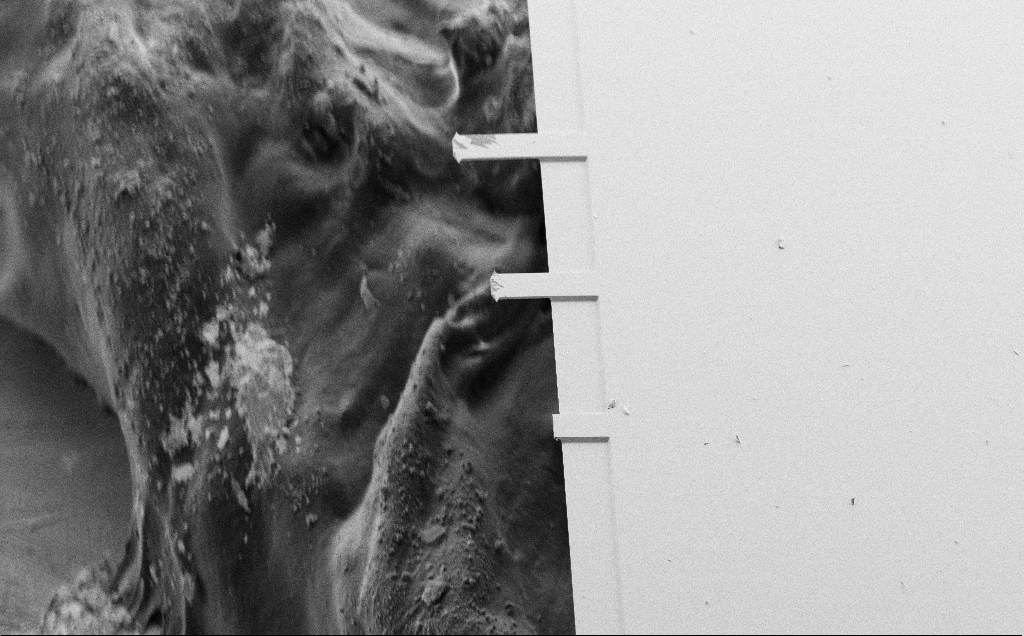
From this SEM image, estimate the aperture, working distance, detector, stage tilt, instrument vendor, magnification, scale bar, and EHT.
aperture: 30 µm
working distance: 3 mm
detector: SE2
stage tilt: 44.2°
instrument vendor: Zeiss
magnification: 0.259 K X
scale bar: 100000 nm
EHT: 10 kV